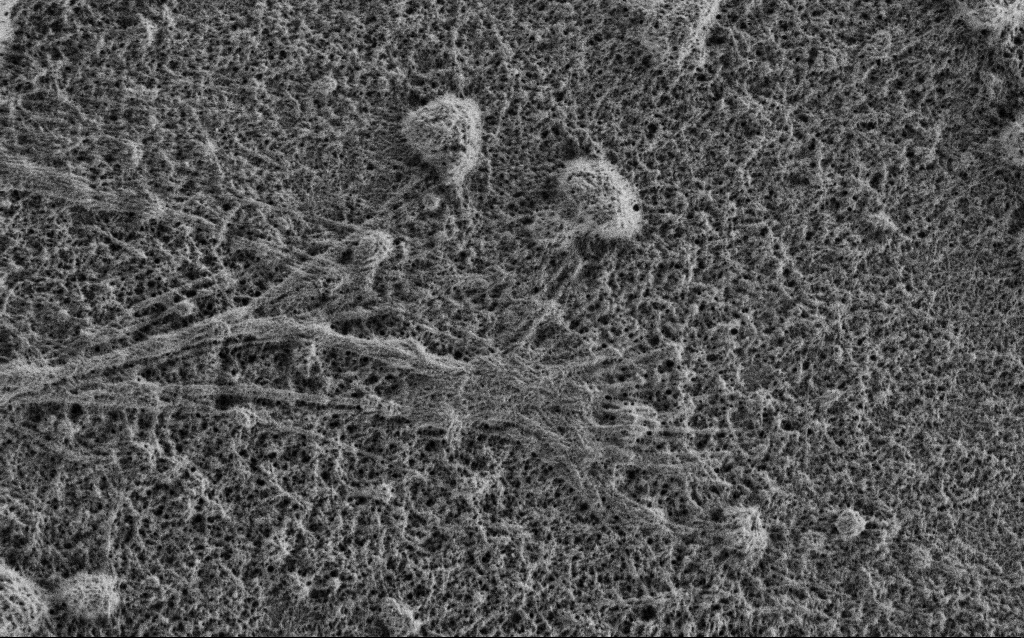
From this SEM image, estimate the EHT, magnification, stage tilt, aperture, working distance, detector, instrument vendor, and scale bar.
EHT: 0.9 kV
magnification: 15 K X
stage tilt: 0°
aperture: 30 µm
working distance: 3.4 mm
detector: SE2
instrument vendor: Zeiss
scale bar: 1000 nm